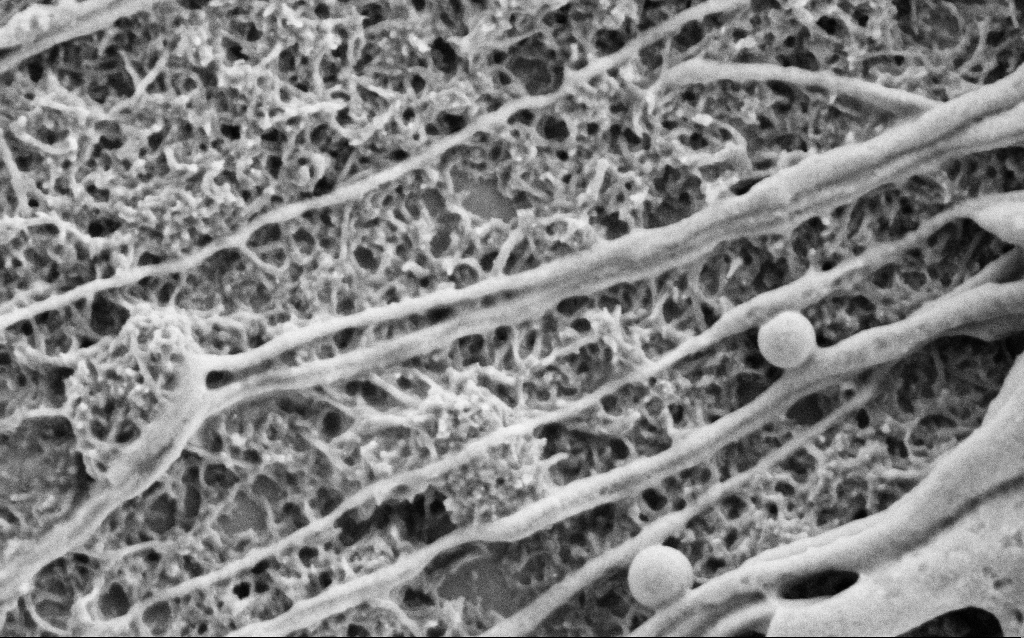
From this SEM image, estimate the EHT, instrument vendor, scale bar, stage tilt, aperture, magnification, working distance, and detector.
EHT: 2 kV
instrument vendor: Zeiss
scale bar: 1000 nm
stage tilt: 0°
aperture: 30 µm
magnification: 50 K X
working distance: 6.8 mm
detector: SE2